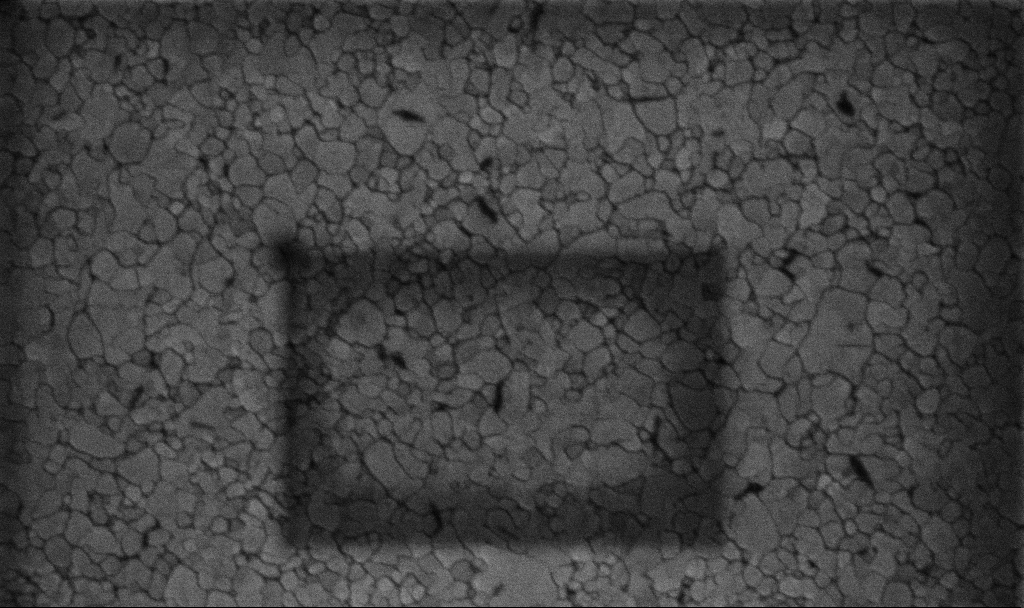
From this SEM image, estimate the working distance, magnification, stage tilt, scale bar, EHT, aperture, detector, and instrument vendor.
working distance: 3.1 mm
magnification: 100.15 K X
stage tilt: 0°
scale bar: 200 nm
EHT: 5 kV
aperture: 30 µm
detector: InLens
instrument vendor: Zeiss